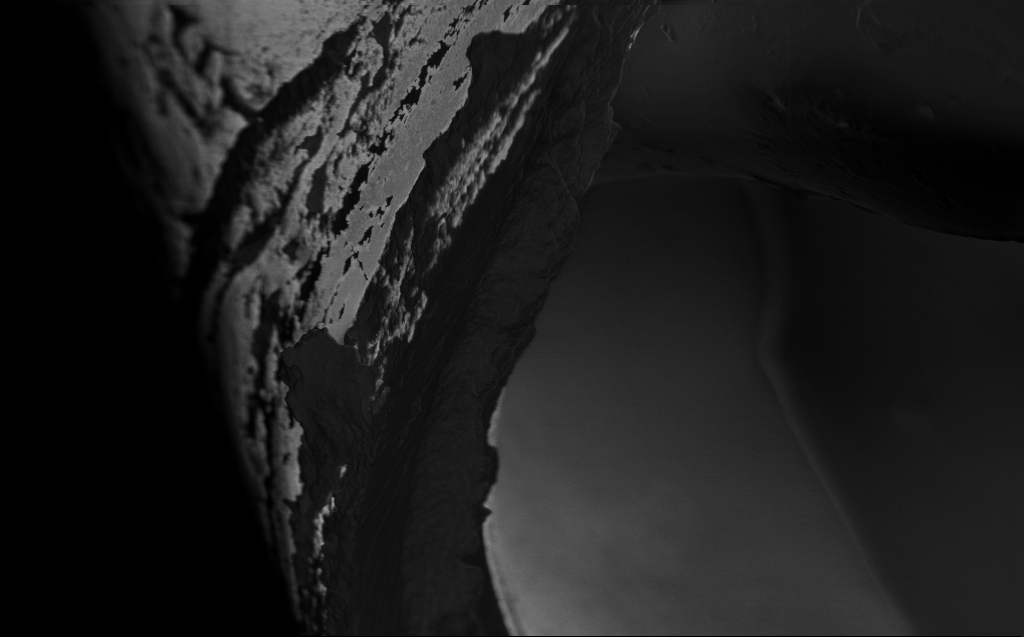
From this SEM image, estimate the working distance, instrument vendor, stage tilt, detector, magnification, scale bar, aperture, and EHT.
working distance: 3 mm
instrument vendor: Zeiss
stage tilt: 45°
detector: InLens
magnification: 10.64 K X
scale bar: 2000 nm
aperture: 30 µm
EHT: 1 kV